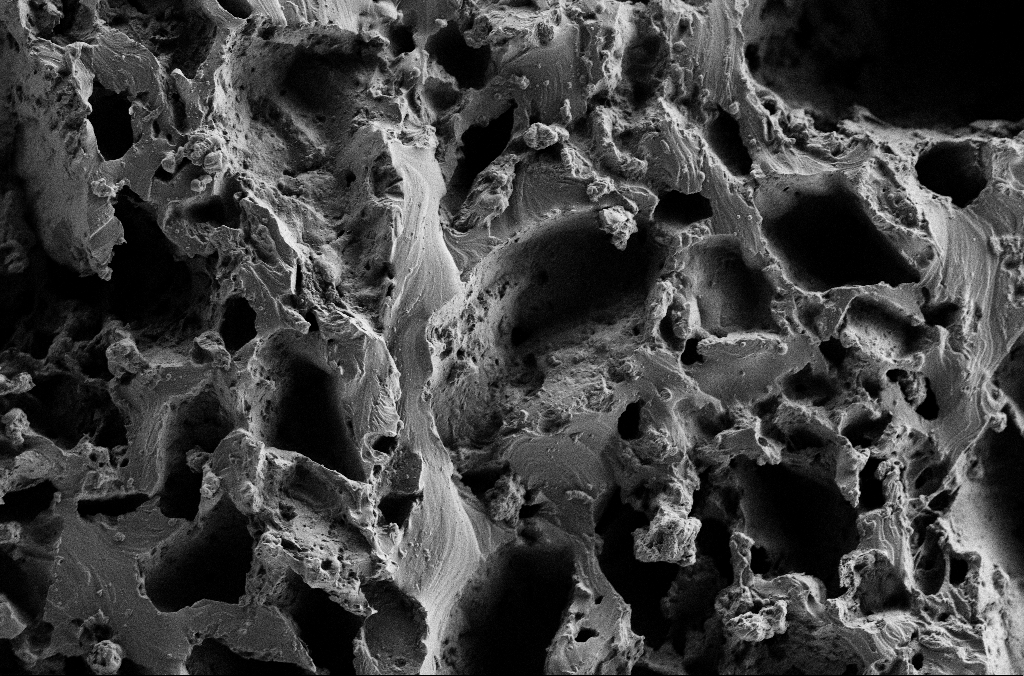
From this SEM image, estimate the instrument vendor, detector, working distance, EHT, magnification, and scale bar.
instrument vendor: Zeiss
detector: SE2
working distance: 3 mm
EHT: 2 kV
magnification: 1 K X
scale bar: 20000 nm